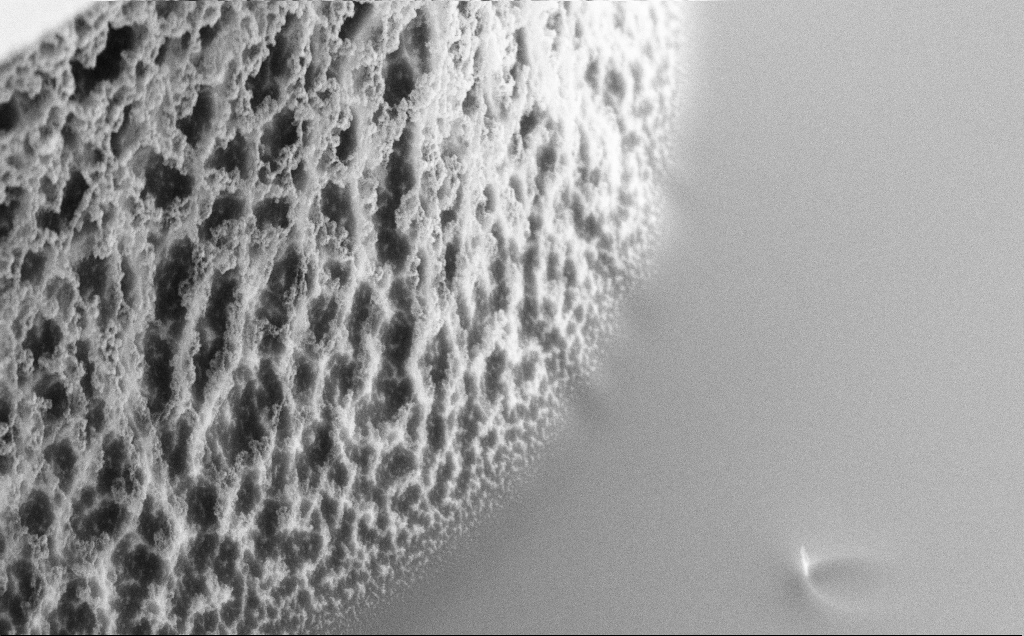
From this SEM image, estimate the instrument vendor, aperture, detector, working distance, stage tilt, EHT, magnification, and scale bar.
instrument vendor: Zeiss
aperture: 30 µm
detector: SE2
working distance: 8 mm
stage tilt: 45°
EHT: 5 kV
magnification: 24.4 K X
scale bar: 2000 nm